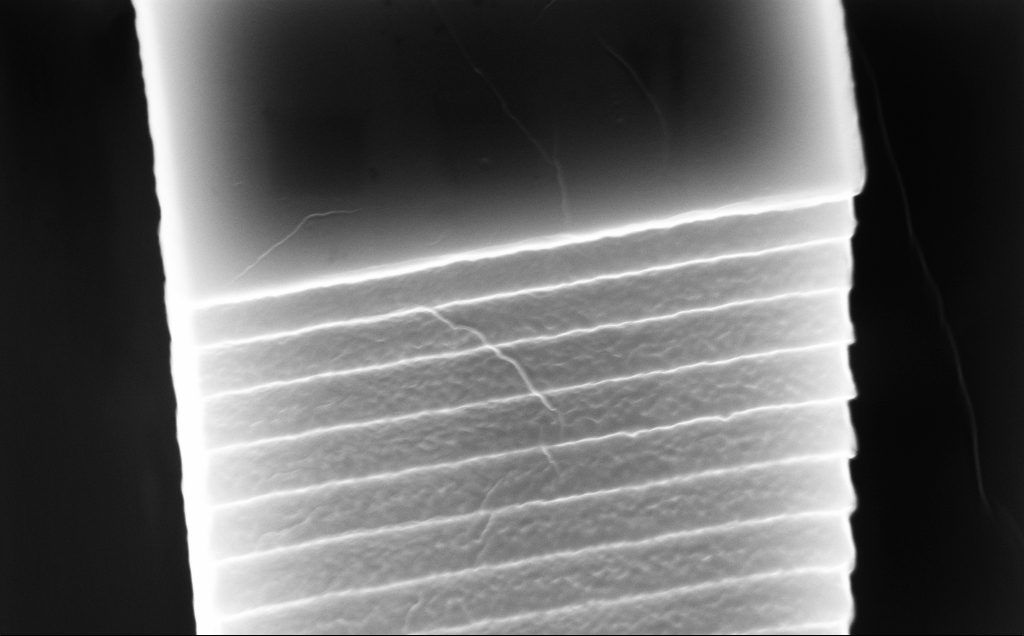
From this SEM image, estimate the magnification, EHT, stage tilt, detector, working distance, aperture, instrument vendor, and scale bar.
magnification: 53.29 K X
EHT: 10 kV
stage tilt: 45°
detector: InLens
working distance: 5 mm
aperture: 30 µm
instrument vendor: Zeiss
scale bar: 1000 nm